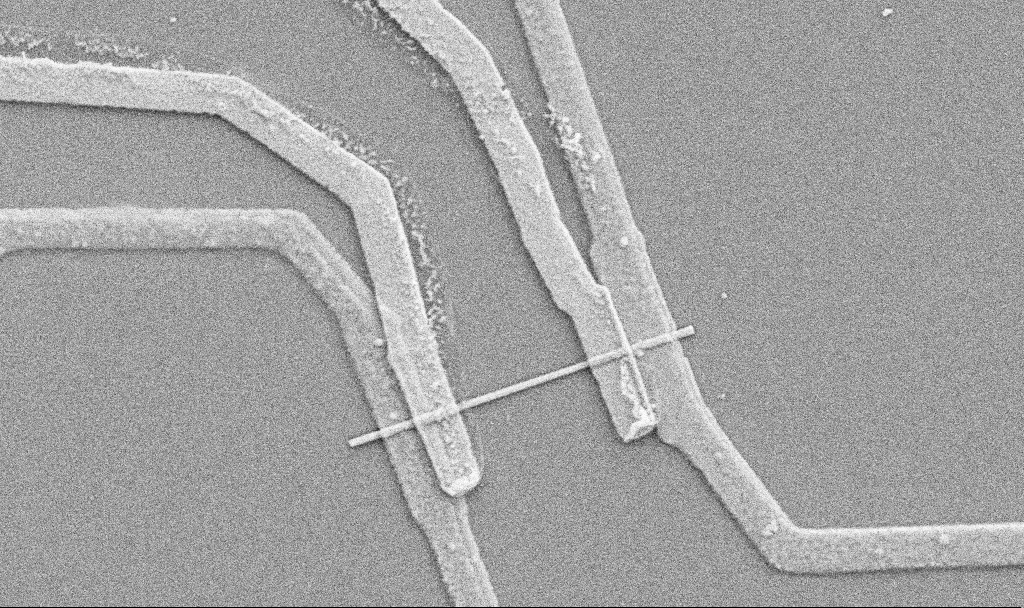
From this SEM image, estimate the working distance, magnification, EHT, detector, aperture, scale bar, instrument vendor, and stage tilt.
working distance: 10.7 mm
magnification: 20 K X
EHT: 5 kV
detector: SE2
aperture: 30 µm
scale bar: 1000 nm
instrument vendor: Zeiss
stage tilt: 0°